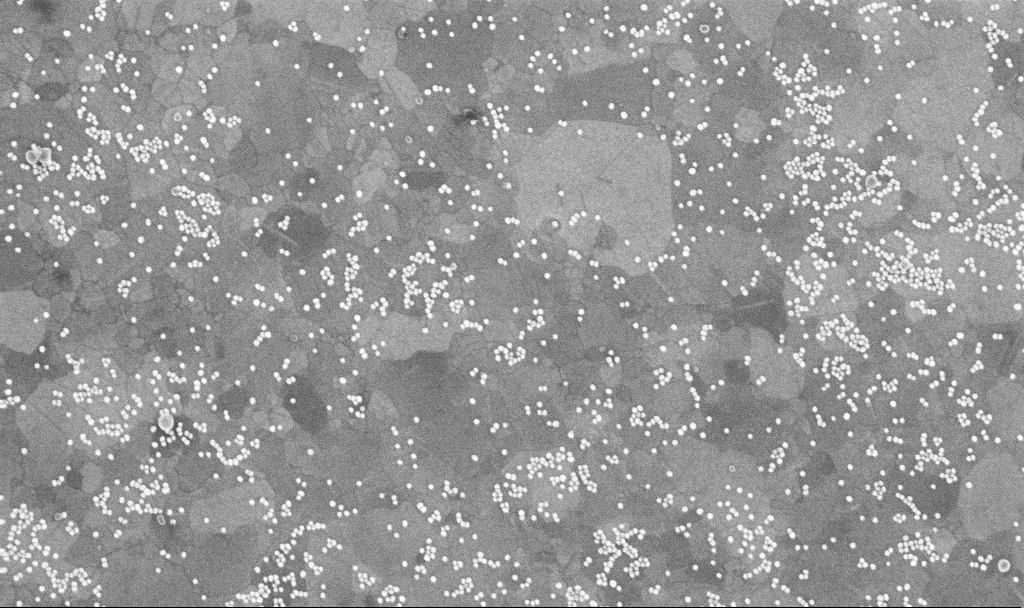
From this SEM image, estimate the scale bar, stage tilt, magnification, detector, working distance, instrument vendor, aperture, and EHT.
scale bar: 200 nm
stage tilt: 0°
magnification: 100 K X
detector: InLens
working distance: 3.7 mm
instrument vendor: Zeiss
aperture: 30 µm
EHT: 10 kV